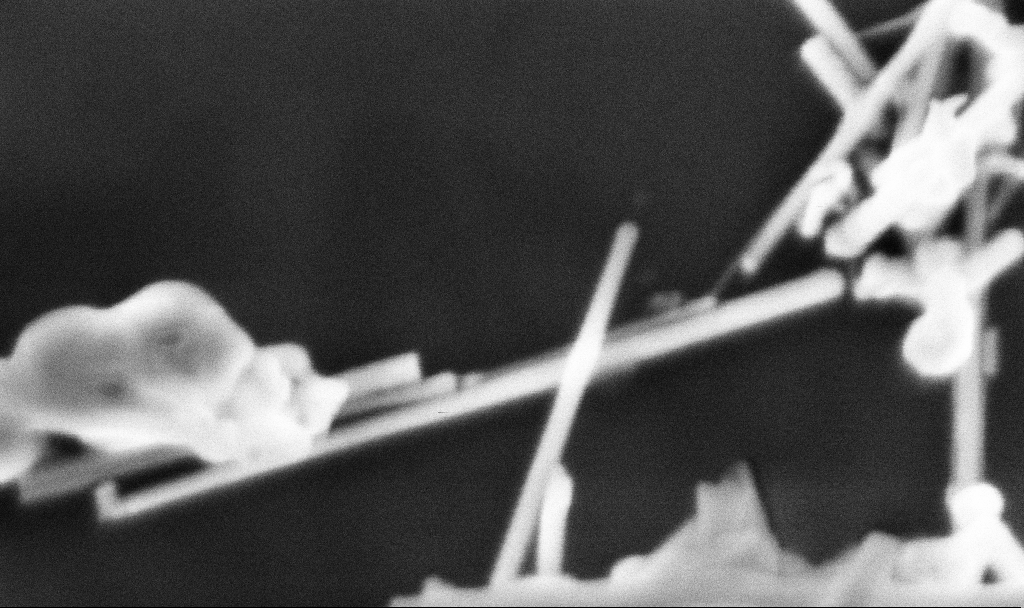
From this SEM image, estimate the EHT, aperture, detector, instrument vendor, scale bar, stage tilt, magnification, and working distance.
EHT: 3 kV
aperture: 30 µm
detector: InLens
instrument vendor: Zeiss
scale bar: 200 nm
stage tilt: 0°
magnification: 222.86 K X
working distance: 3.3 mm